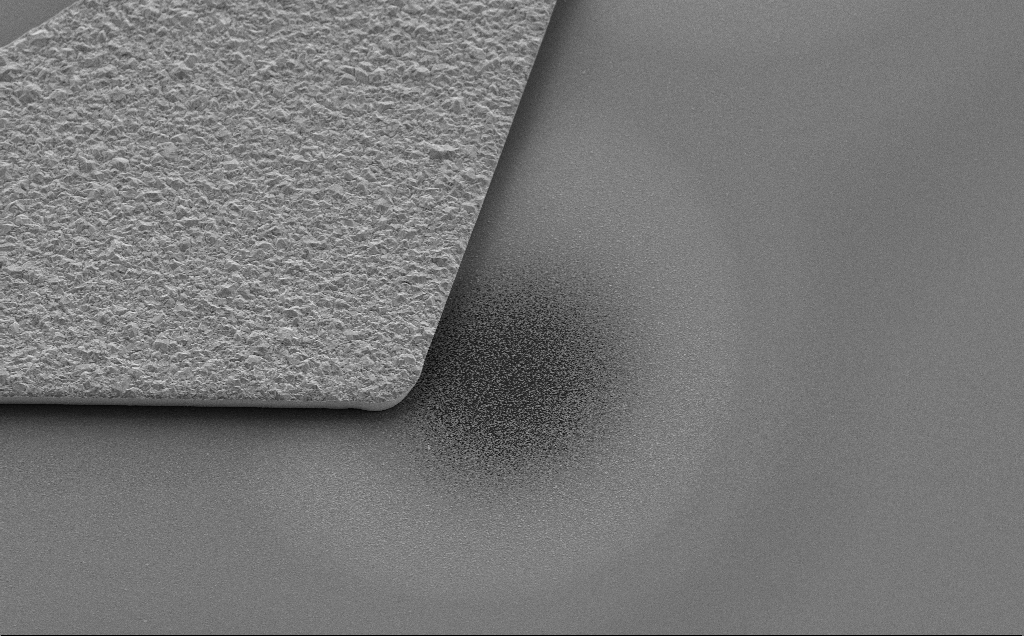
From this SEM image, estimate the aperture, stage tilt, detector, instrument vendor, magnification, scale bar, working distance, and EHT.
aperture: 30 µm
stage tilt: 30°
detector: SE2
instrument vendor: Zeiss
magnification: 6.04 K X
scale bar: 10000 nm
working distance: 9 mm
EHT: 5 kV